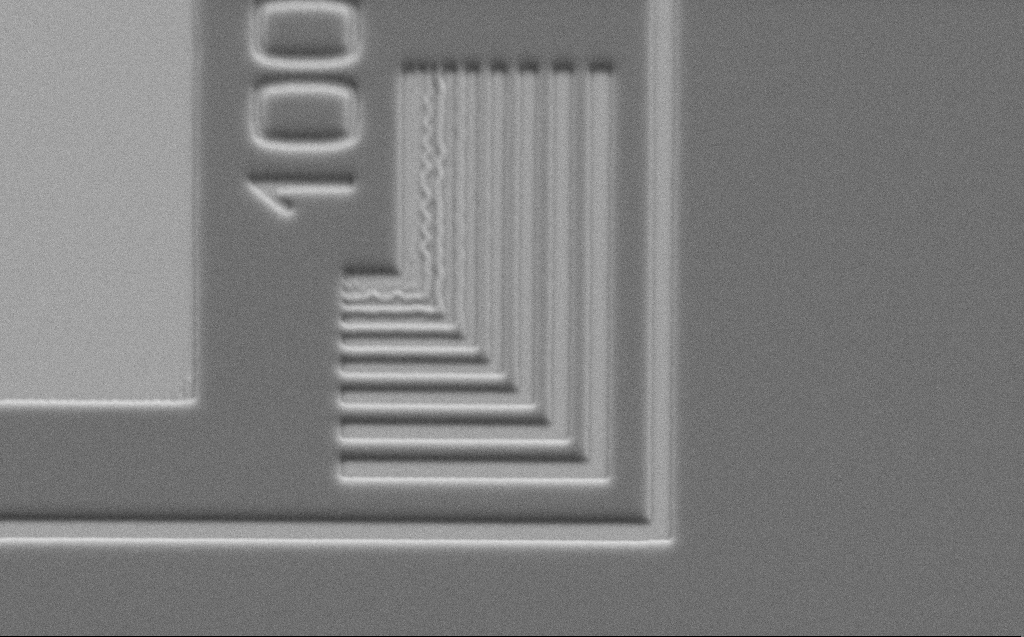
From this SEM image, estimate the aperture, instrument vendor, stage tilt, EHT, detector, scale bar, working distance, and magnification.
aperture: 30 µm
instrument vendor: Zeiss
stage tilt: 45°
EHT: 5 kV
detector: SE2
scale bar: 2000 nm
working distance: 5 mm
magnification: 7.72 K X